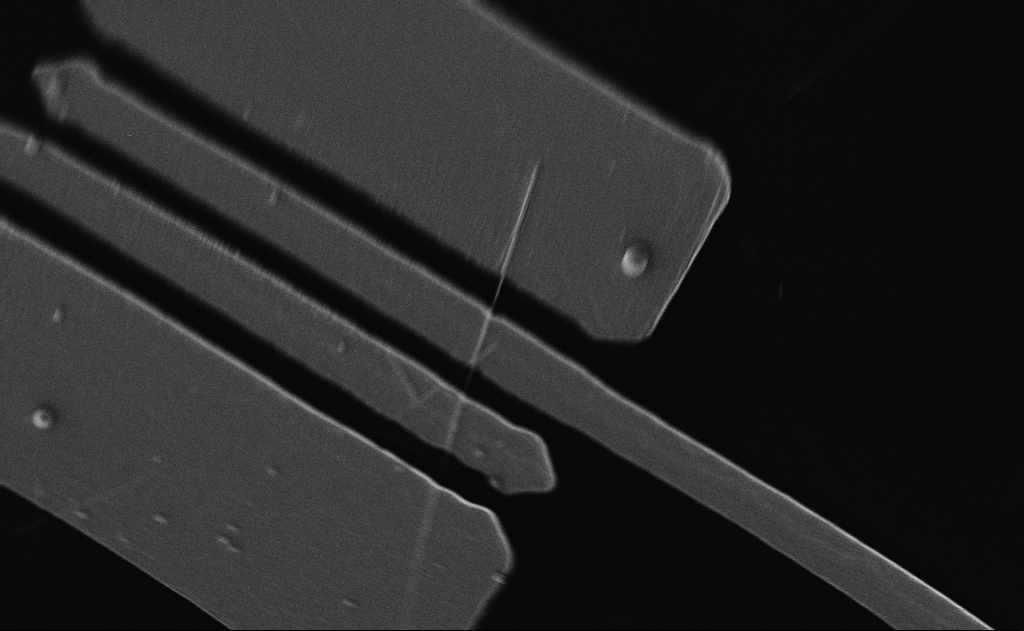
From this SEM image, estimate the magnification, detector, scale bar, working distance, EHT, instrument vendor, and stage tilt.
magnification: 8 K X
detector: InLens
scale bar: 2000 nm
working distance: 21 mm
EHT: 5 kV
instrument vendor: Zeiss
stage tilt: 0°